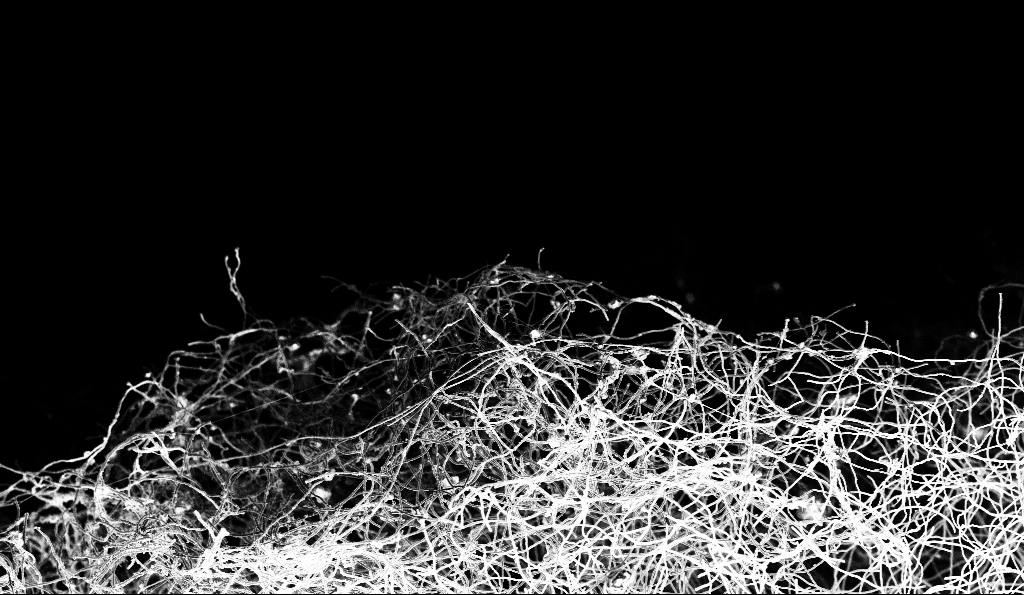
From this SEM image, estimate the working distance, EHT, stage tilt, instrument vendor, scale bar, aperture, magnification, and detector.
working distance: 5 mm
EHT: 3 kV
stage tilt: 0°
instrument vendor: Zeiss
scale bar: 20000 nm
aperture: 30 µm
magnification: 1 K X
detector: InLens